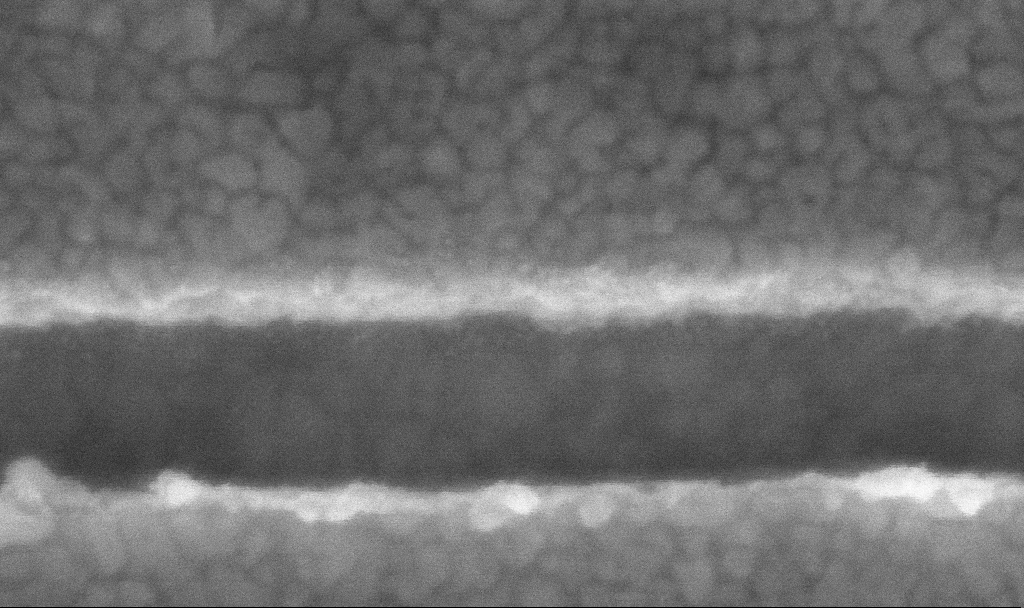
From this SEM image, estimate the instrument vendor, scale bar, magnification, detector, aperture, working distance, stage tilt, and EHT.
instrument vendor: Zeiss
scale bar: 100 nm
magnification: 553.86 K X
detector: InLens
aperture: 60 µm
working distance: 3.8 mm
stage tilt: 0°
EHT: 5 kV